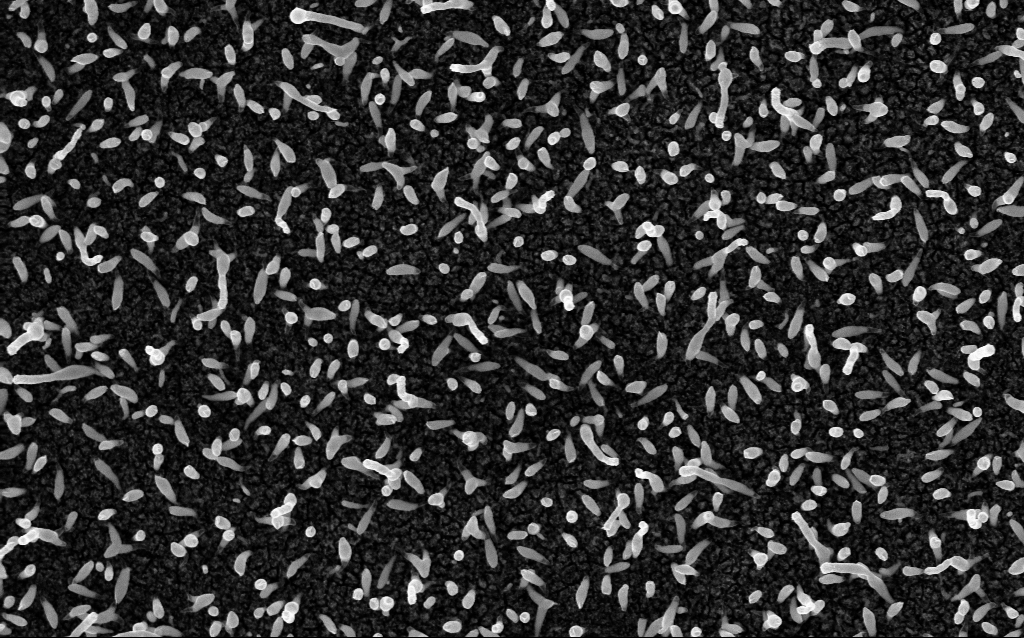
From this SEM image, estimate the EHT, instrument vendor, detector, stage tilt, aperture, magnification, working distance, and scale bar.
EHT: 5 kV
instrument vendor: Zeiss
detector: InLens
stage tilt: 0°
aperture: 30 µm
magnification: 50 K X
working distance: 2.1 mm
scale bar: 1000 nm